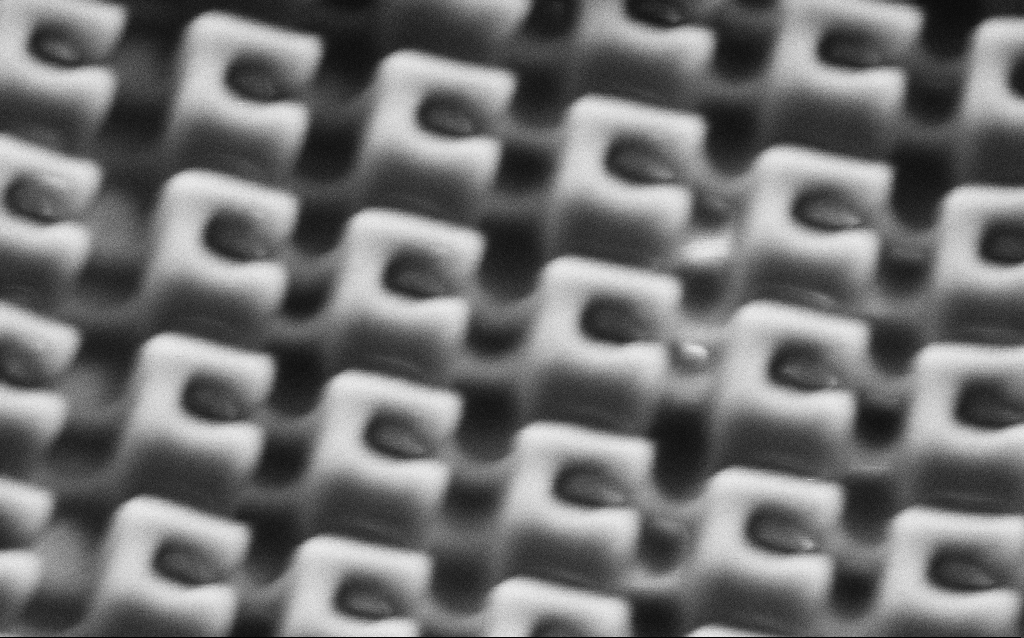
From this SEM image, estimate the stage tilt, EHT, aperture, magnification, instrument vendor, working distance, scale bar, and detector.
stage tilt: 0°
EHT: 1.5 kV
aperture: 30 µm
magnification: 155.84 K X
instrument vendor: Zeiss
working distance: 5.9 mm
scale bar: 200 nm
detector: SE2